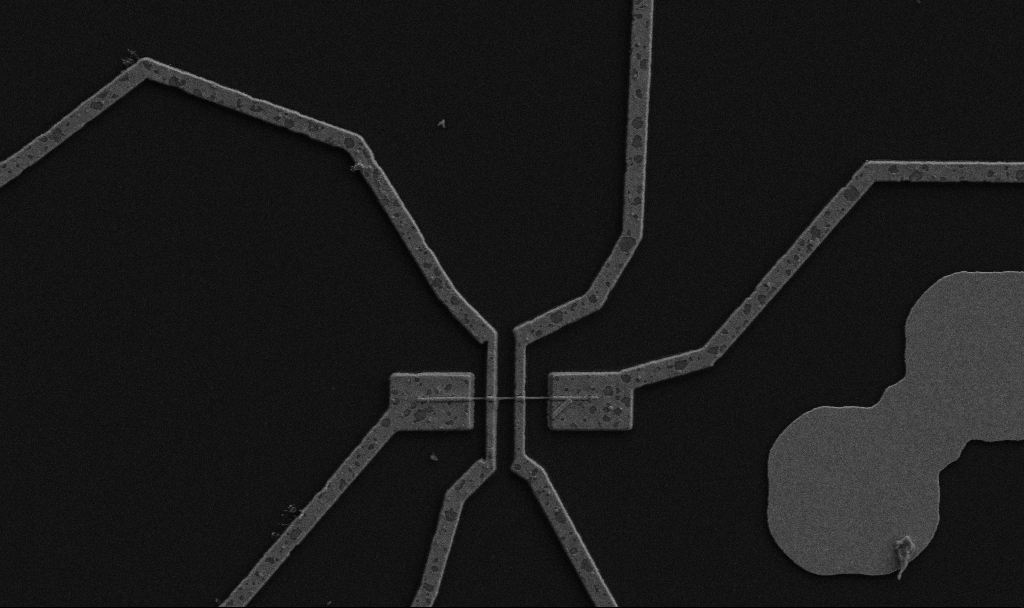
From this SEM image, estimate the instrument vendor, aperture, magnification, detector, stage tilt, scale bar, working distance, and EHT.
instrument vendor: Zeiss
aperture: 30 µm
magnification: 10 K X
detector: SE2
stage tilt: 0°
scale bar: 2000 nm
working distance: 9.7 mm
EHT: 5 kV